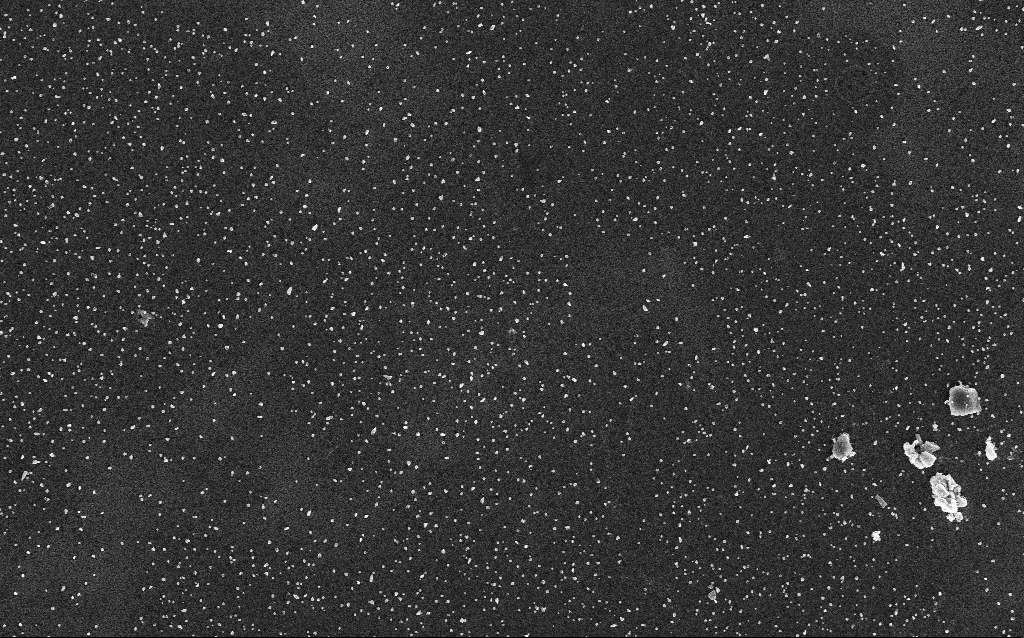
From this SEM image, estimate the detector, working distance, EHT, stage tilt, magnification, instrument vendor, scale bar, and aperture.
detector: InLens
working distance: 1.9 mm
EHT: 5 kV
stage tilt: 0°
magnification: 10 K X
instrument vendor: Zeiss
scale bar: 2000 nm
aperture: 30 µm